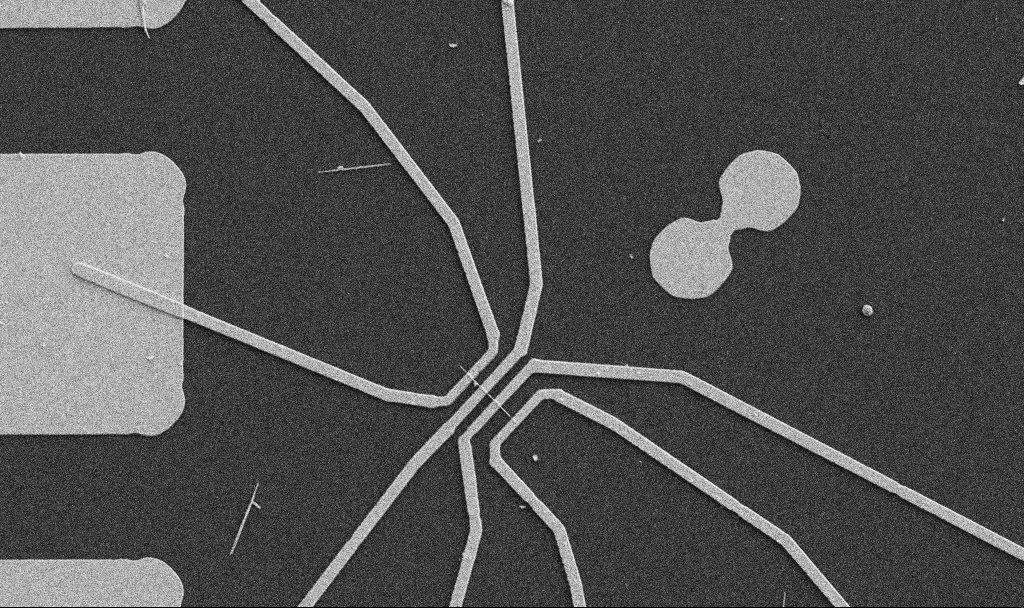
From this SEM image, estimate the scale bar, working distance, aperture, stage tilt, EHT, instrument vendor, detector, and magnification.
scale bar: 10000 nm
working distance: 10.7 mm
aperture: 30 µm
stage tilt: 0°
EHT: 5 kV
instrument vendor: Zeiss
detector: SE2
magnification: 5 K X